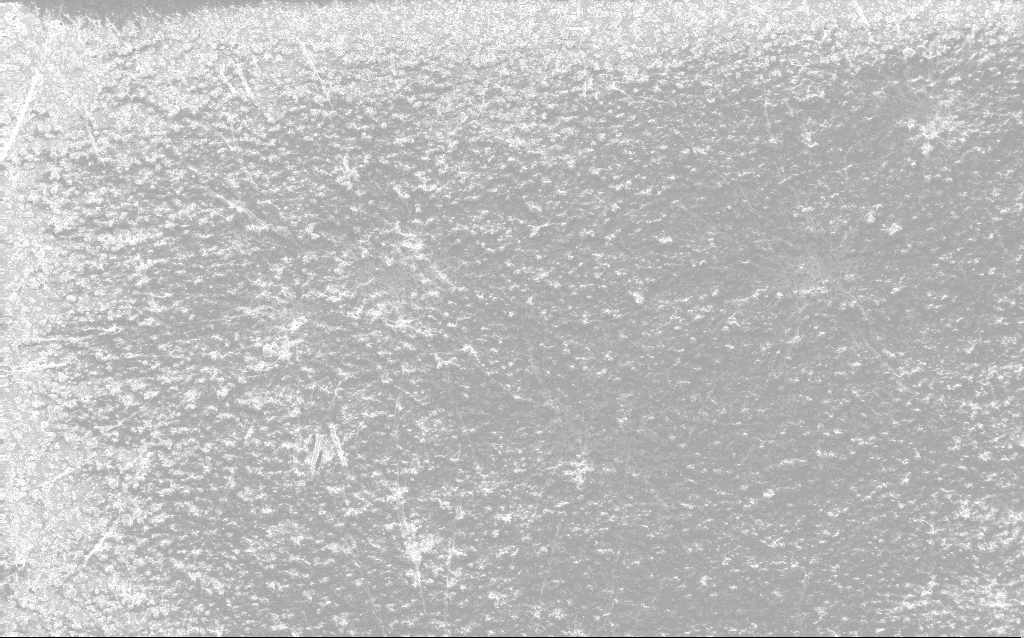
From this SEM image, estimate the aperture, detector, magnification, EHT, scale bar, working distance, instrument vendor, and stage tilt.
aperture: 30 µm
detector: InLens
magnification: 0.235 K X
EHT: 10 kV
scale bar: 100000 nm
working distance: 2.7 mm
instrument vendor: Zeiss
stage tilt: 0°